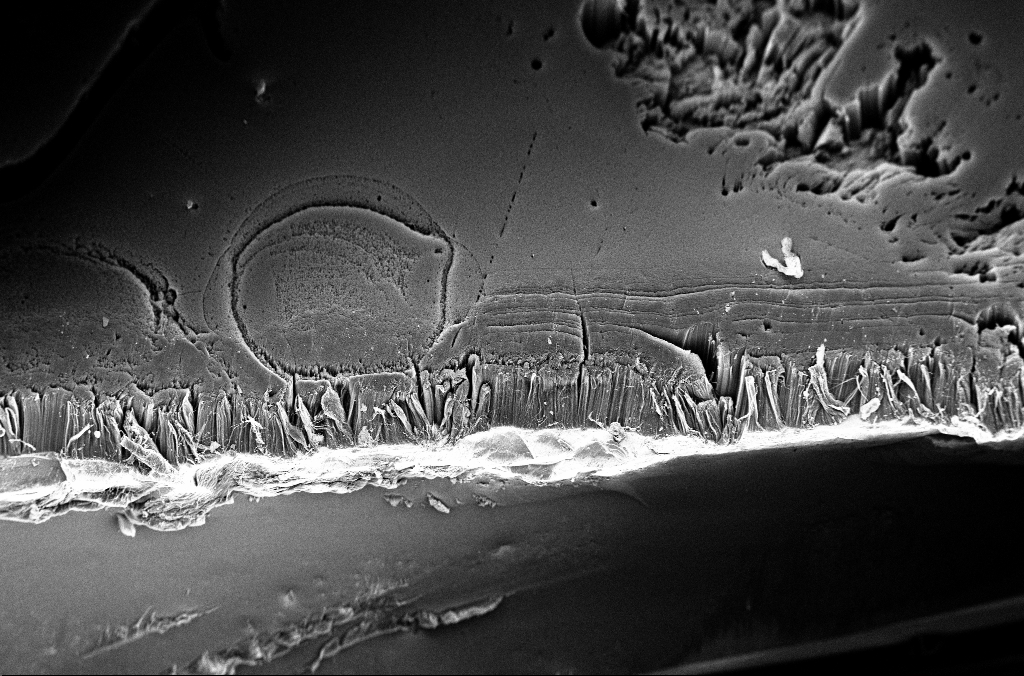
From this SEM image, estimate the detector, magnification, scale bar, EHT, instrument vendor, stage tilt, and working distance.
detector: InLens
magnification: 0.2 K X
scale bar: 100000 nm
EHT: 3 kV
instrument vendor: Zeiss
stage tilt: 45°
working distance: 3.3 mm